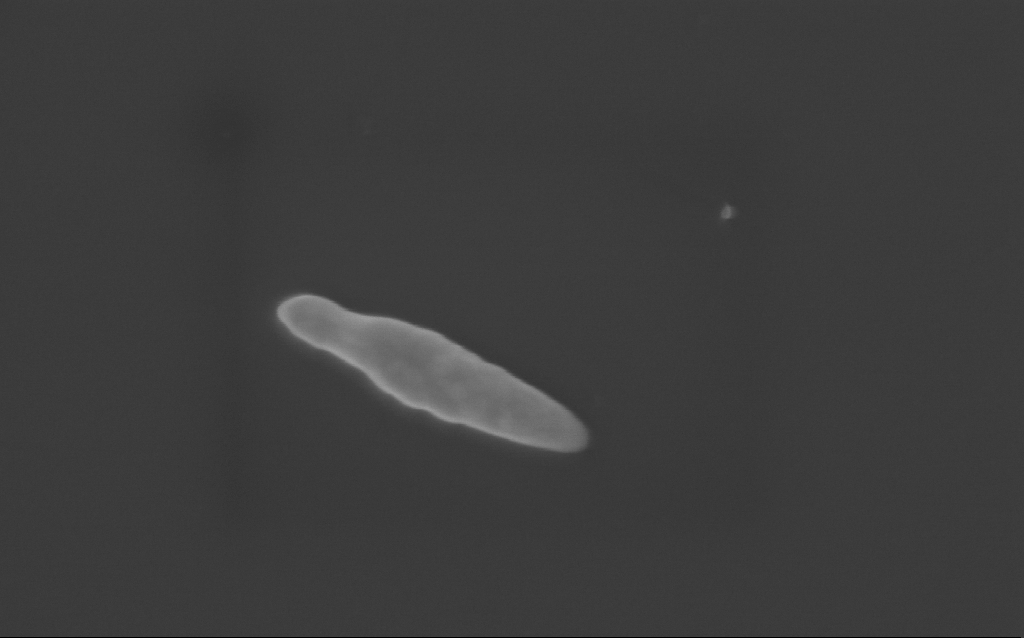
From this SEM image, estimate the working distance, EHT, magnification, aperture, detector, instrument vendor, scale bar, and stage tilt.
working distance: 3 mm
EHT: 3 kV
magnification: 132.71 K X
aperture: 30 µm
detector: InLens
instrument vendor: Zeiss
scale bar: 200 nm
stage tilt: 0°